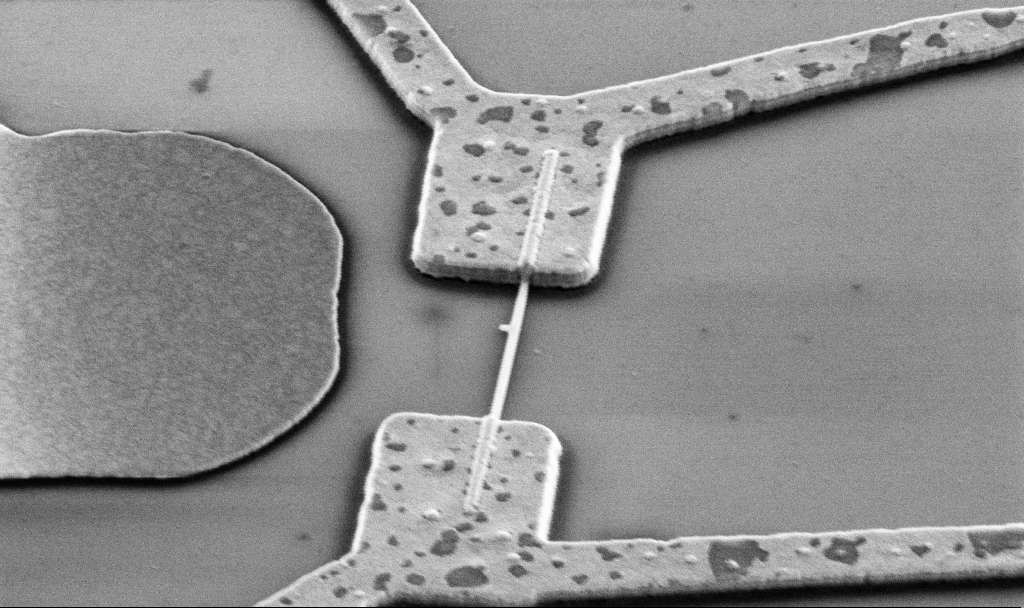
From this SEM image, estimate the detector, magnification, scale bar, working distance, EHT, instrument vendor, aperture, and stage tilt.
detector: SE2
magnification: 30 K X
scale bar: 1000 nm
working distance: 15.7 mm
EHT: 5 kV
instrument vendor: Zeiss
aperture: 30 µm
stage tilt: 45°